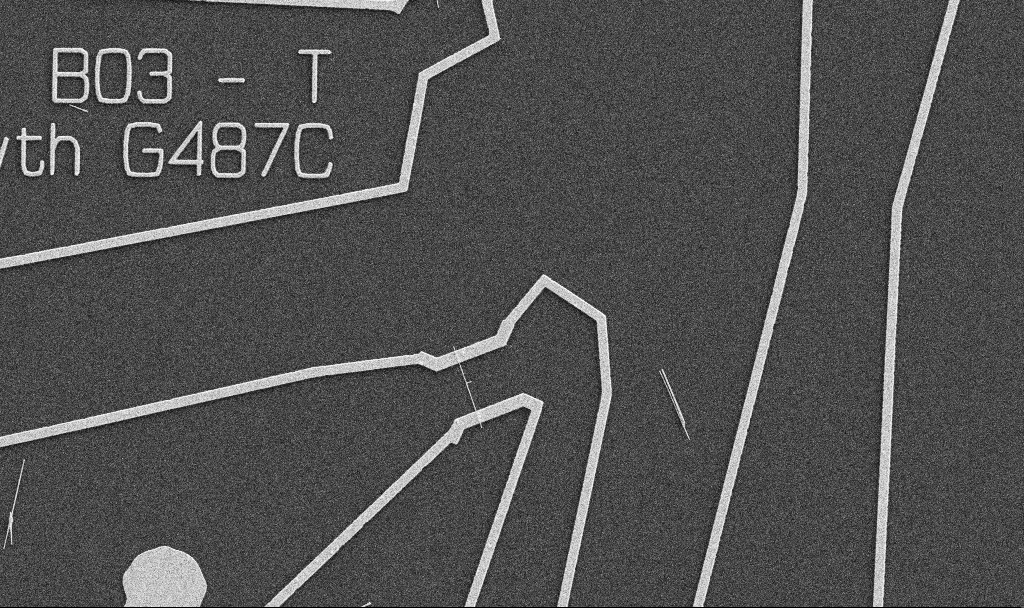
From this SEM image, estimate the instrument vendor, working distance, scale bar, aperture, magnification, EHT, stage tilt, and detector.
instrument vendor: Zeiss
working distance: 10.7 mm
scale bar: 10000 nm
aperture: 30 µm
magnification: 5 K X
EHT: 5 kV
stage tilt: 0°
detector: SE2